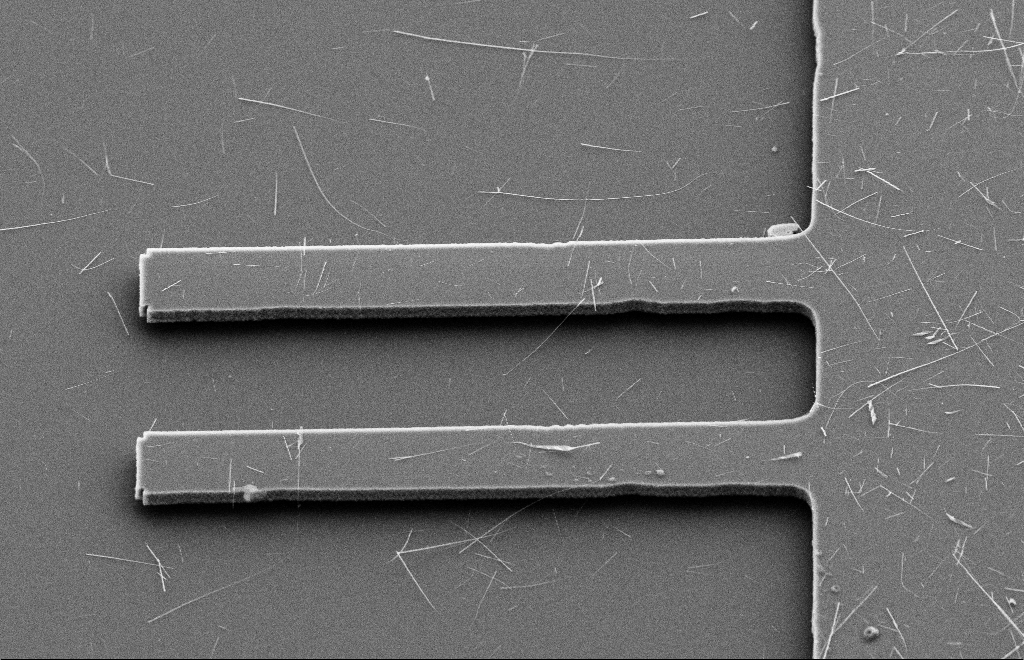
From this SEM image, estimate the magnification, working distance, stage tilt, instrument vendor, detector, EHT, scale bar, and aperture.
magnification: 2.5 K X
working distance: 9 mm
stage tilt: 39.8°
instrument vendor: Zeiss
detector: SE2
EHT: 10 kV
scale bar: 10000 nm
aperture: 20 µm